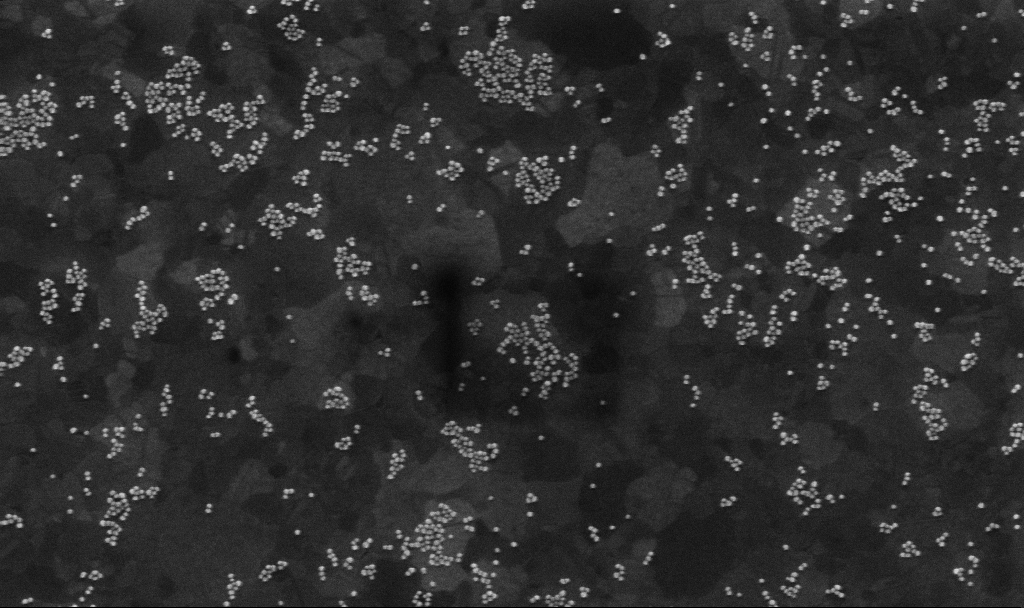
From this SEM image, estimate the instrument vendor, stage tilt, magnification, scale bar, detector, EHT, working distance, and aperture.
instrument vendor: Zeiss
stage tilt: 0°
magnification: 103.66 K X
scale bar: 200 nm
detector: InLens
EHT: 10 kV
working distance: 3.8 mm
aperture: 30 µm